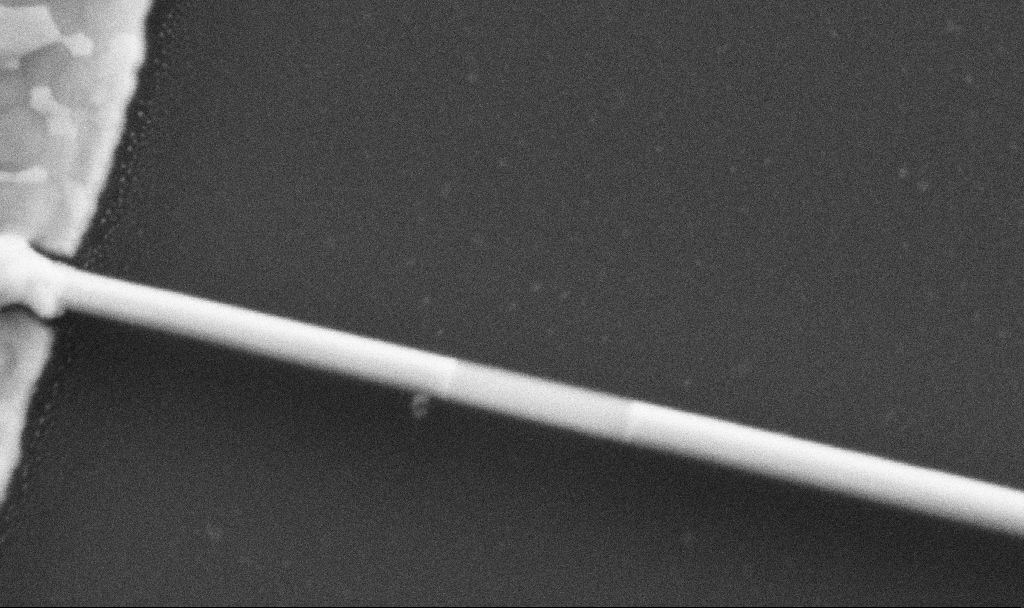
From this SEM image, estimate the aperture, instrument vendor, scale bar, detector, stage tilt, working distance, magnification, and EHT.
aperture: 30 µm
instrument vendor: Zeiss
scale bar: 100 nm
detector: SE2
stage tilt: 0°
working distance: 8.7 mm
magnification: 200 K X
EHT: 5 kV